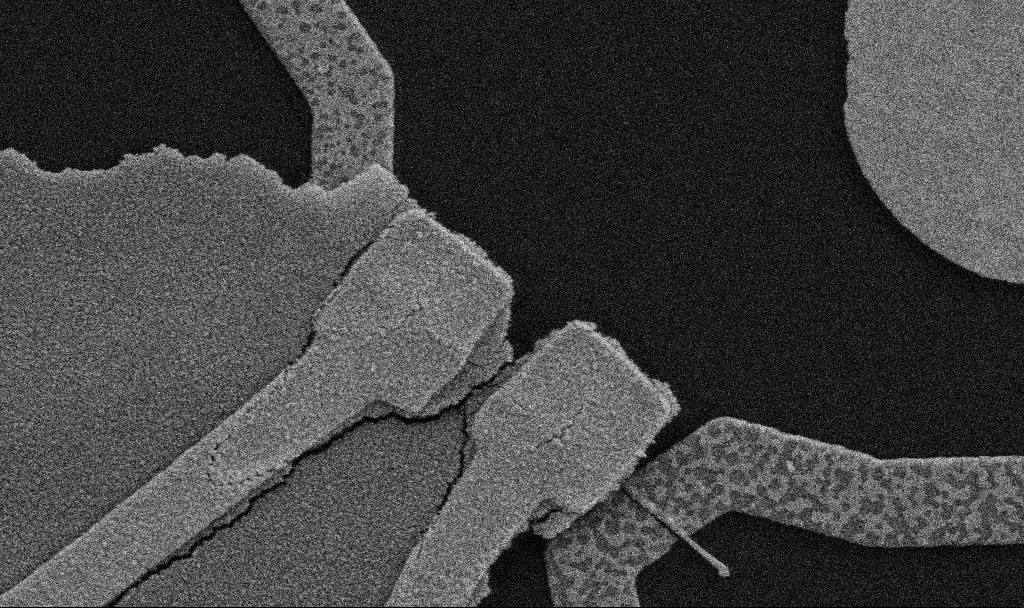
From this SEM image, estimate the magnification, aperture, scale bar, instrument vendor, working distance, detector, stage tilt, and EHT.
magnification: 30 K X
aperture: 30 µm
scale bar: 1000 nm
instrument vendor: Zeiss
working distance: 10.7 mm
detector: SE2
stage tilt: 0°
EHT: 5 kV